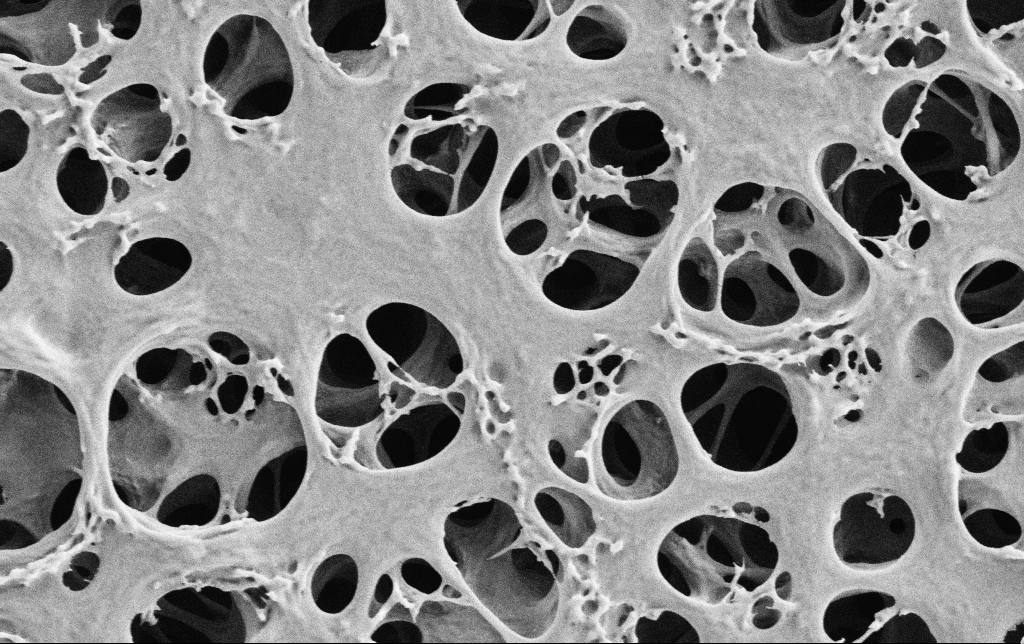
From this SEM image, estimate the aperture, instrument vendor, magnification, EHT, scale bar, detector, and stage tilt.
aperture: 30 µm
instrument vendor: Zeiss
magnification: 25 K X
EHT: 2 kV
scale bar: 1000 nm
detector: SE2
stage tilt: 0°